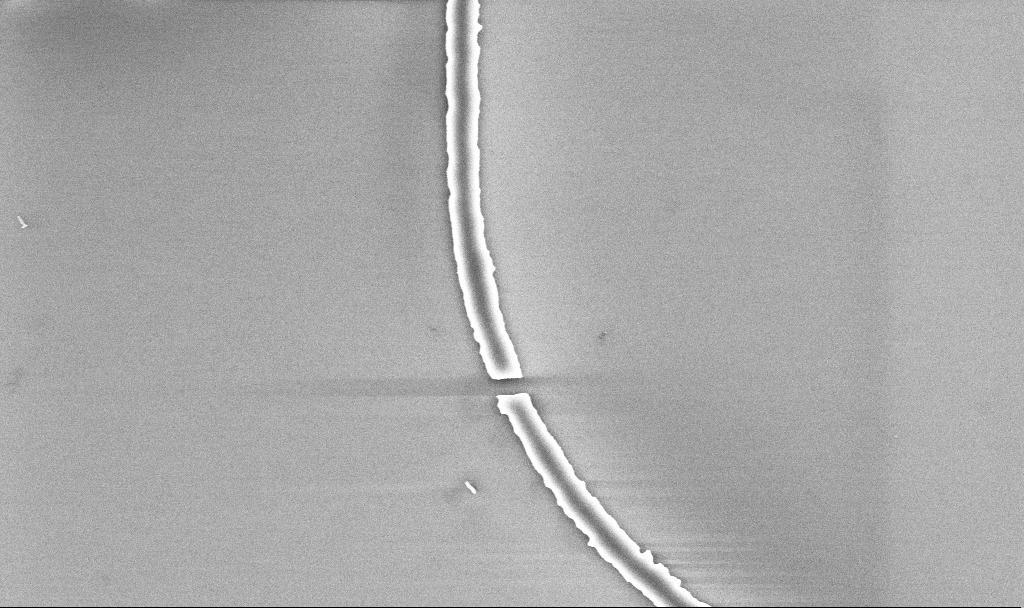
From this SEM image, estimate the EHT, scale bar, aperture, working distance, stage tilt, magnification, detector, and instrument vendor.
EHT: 5 kV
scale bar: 2000 nm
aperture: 30 µm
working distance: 5.2 mm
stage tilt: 0°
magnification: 21.1 K X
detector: InLens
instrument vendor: Zeiss